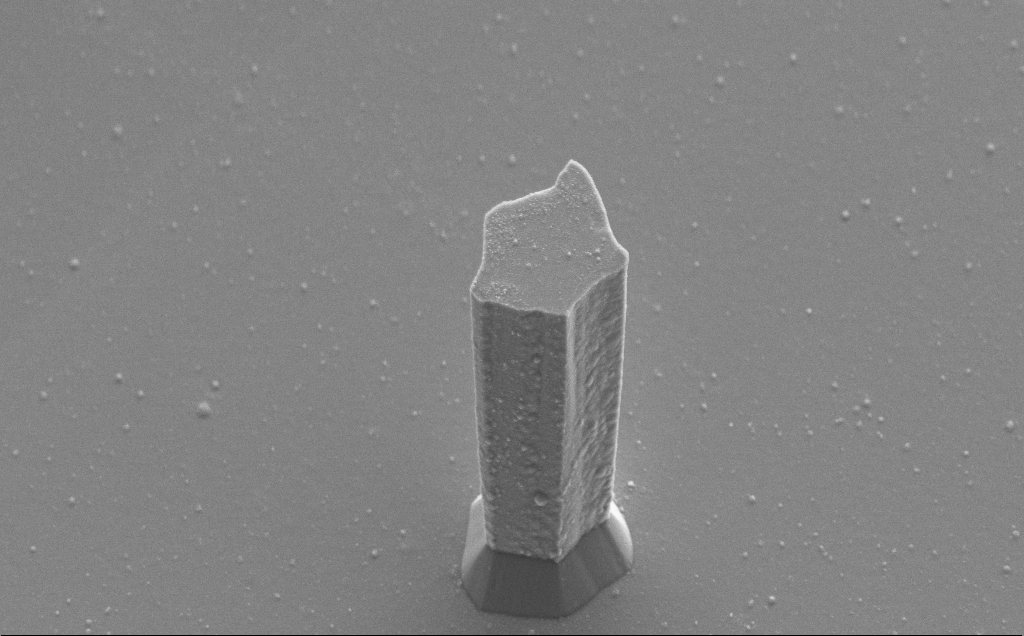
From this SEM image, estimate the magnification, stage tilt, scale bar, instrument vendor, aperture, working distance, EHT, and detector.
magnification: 5.39 K X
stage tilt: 46.4°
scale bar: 10000 nm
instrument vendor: Zeiss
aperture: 30 µm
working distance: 10 mm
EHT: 5 kV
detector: SE2